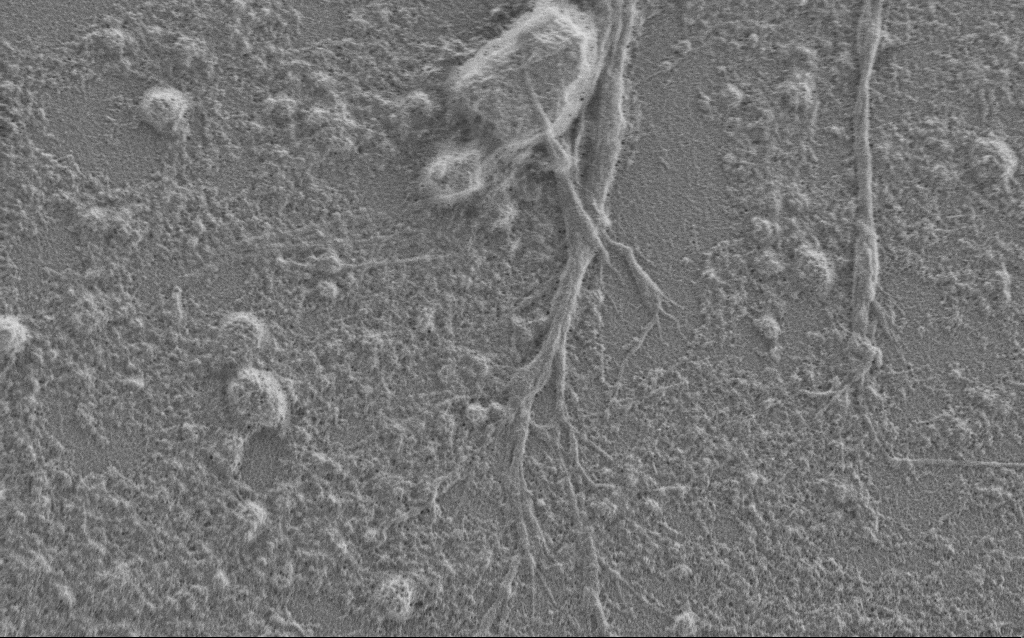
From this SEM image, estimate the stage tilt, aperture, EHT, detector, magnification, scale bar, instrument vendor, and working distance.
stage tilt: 0°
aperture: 30 µm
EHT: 1 kV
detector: SE2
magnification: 7.5 K X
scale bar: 2000 nm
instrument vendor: Zeiss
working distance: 6 mm